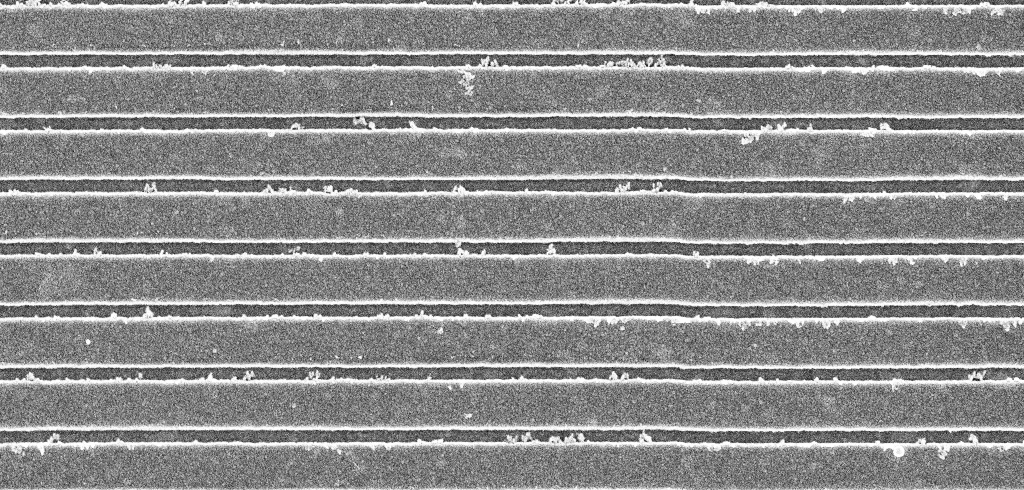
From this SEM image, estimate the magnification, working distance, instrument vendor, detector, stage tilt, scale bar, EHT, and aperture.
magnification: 38.57 K X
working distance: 5.3 mm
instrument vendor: Zeiss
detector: InLens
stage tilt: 0°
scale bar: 1000 nm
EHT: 5 kV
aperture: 30 µm